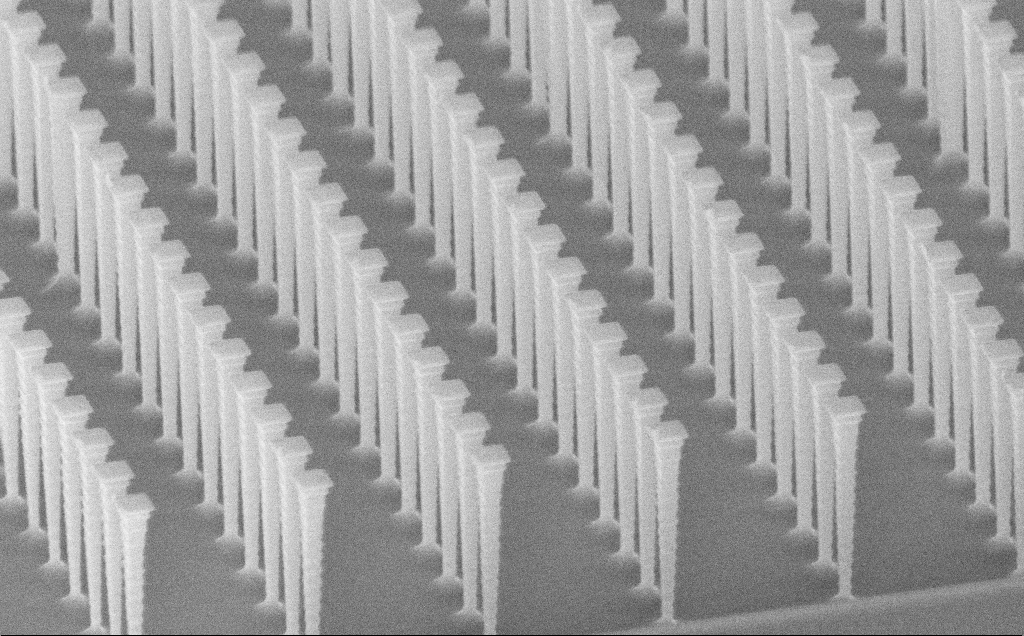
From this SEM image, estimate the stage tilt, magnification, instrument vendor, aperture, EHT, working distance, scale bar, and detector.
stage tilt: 62°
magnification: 13.67 K X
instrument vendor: Zeiss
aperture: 30 µm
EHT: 10 kV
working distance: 8 mm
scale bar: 1000 nm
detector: InLens